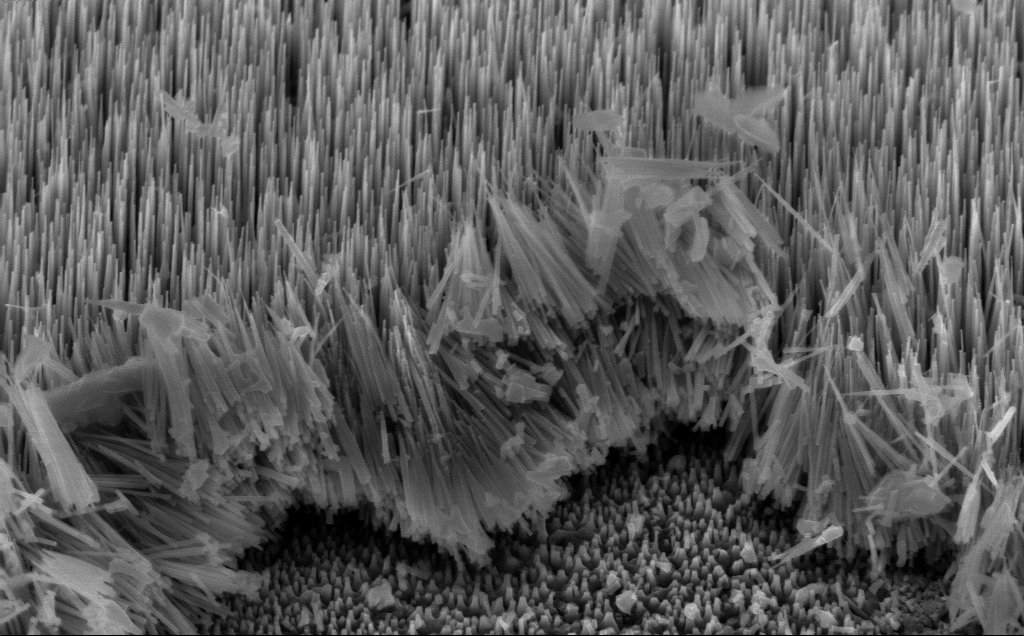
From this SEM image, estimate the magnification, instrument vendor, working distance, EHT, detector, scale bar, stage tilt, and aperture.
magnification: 37.24 K X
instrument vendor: Zeiss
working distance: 5 mm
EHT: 10 kV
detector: InLens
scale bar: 1000 nm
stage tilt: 45°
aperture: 30 µm